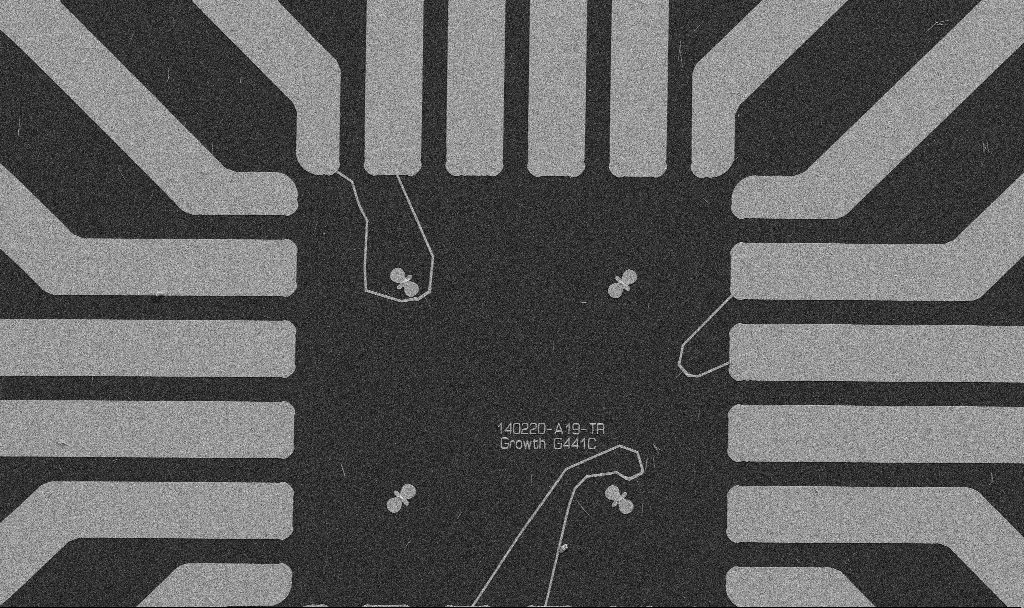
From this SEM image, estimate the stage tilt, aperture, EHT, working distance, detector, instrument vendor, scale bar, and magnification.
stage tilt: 0°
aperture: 30 µm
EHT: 5 kV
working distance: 10.7 mm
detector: SE2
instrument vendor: Zeiss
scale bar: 20000 nm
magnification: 1 K X